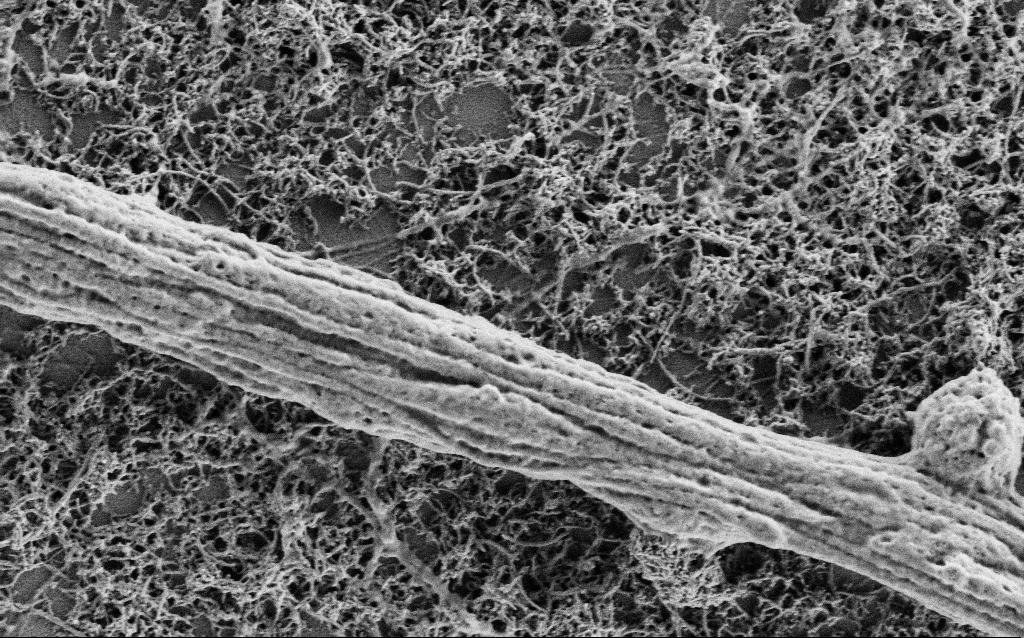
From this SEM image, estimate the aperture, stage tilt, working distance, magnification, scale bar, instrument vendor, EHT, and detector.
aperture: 30 µm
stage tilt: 0°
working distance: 4 mm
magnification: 30 K X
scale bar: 1000 nm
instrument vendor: Zeiss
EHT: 1 kV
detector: SE2